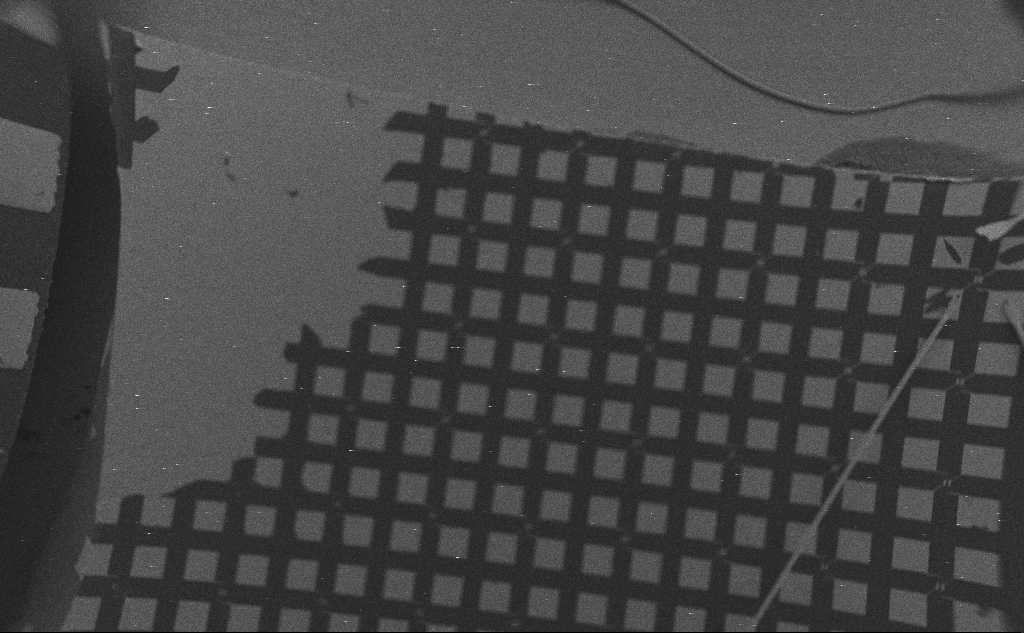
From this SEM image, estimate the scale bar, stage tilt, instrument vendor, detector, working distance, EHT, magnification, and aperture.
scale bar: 1e+06 nm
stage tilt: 0°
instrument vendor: Zeiss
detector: SE2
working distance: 9 mm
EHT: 5 kV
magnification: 0.067 K X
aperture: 30 µm